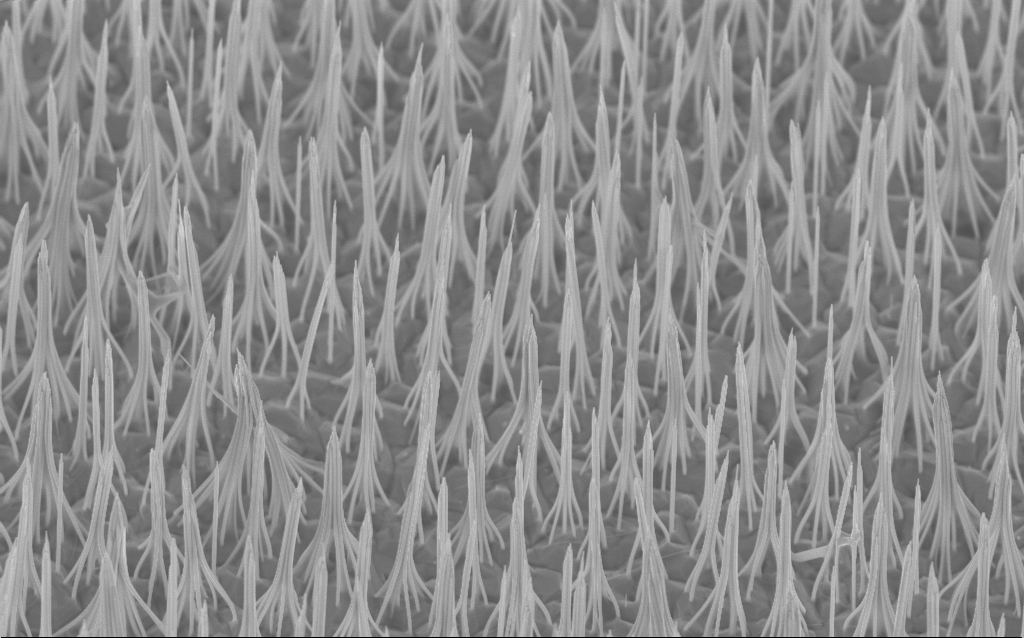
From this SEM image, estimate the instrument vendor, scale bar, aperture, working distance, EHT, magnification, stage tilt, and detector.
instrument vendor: Zeiss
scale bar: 1000 nm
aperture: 30 µm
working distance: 6.3 mm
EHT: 5 kV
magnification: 17.41 K X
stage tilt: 40°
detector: InLens